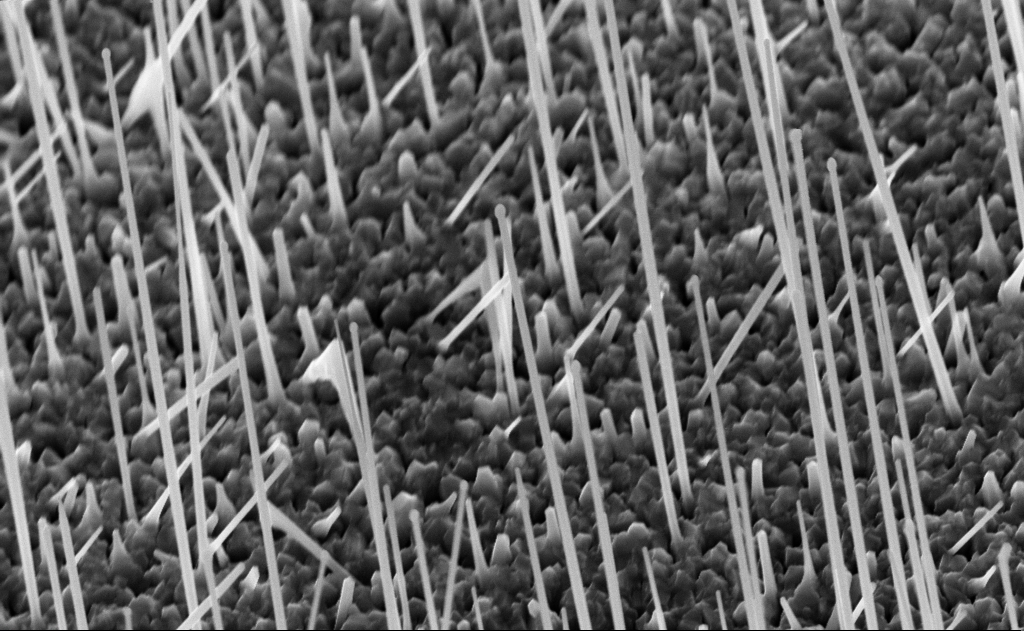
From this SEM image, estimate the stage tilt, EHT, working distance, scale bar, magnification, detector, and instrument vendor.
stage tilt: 0°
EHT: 10 kV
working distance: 8 mm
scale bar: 1000 nm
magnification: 40 K X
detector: InLens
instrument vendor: Zeiss